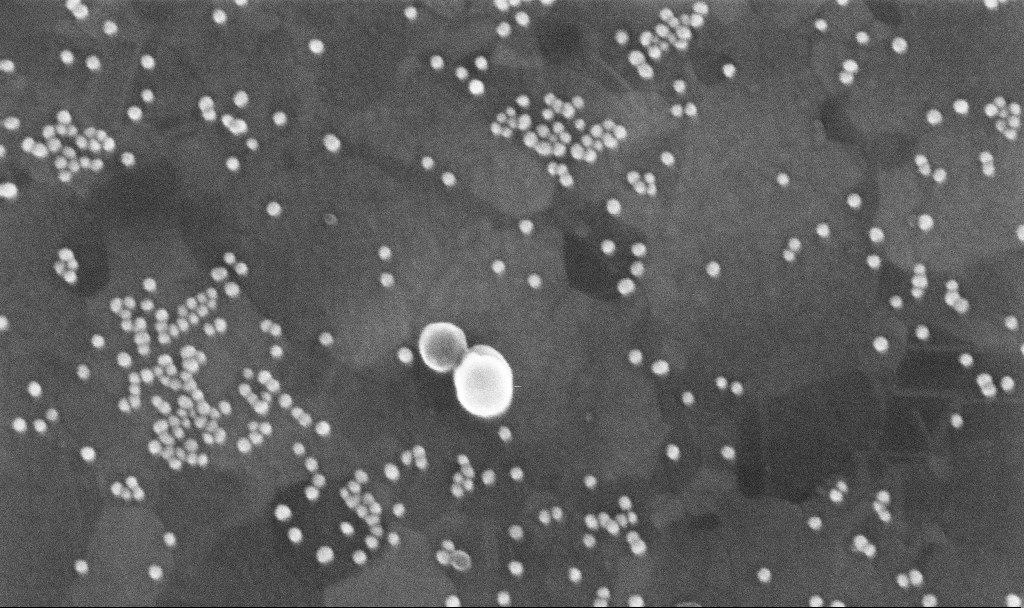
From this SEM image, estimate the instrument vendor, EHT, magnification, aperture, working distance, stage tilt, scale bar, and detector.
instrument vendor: Zeiss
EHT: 10 kV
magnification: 254.91 K X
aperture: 30 µm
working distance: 3.7 mm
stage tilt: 0°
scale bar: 200 nm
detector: InLens